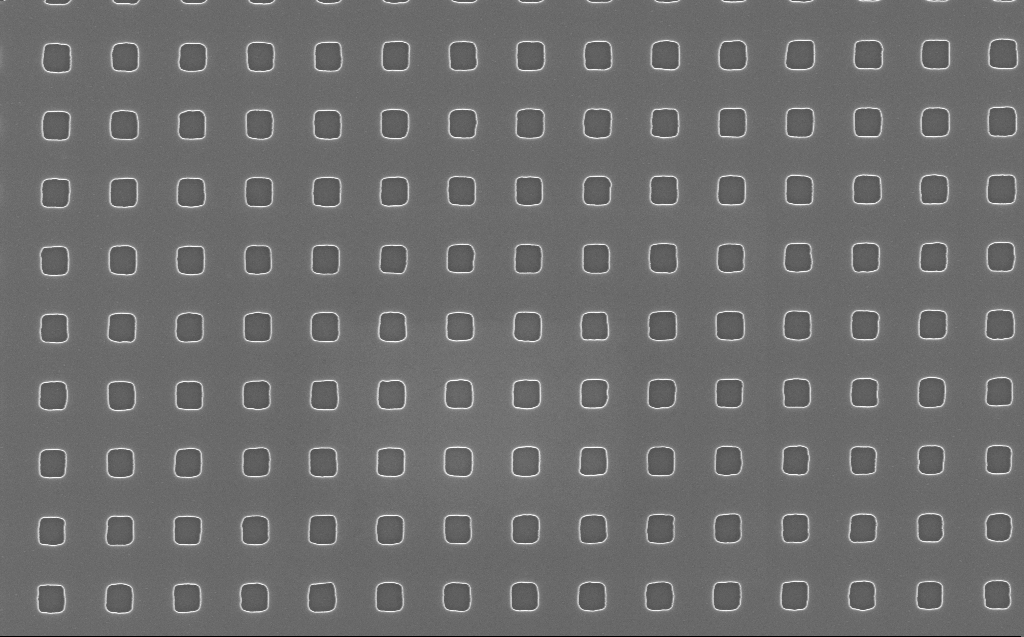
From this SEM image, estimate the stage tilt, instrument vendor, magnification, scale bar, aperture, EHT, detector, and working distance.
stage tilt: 0°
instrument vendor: Zeiss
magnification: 50 K X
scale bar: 1000 nm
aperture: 30 µm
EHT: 10 kV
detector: InLens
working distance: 7 mm